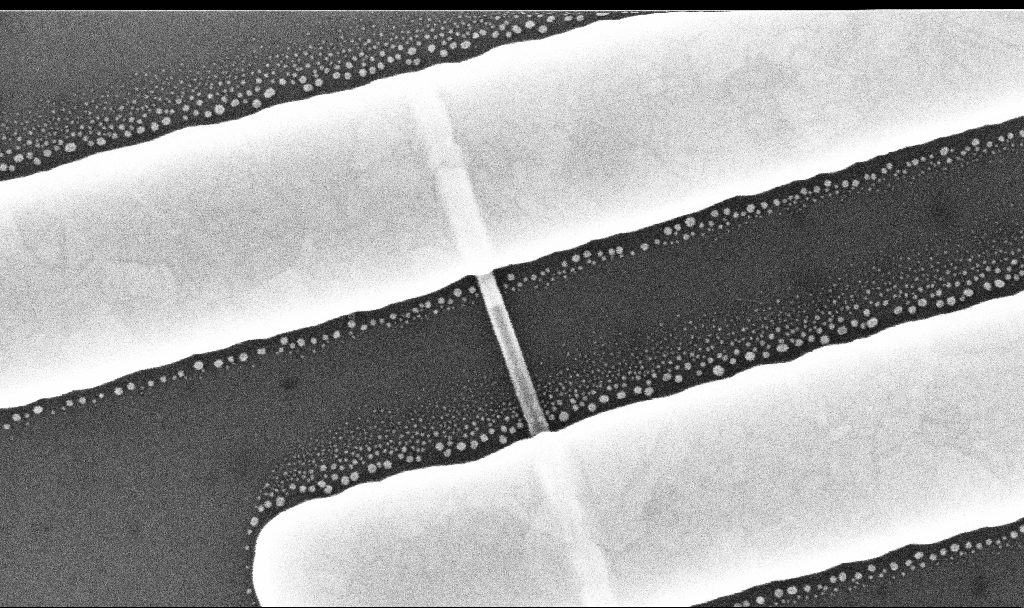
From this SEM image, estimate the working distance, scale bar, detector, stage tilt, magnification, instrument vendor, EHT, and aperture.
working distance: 7 mm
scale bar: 200 nm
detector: InLens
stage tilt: -0°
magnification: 189.11 K X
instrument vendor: Zeiss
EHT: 10 kV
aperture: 30 µm